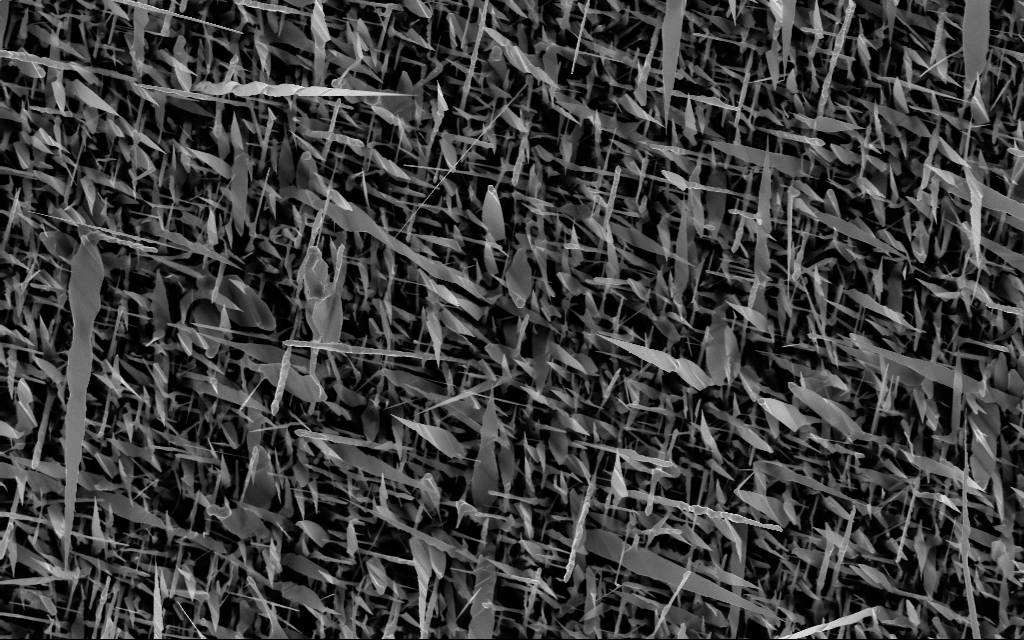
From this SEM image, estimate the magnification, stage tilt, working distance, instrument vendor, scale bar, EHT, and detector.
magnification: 10 K X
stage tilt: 0°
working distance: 6 mm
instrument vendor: Zeiss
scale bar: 2000 nm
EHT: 10 kV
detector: InLens